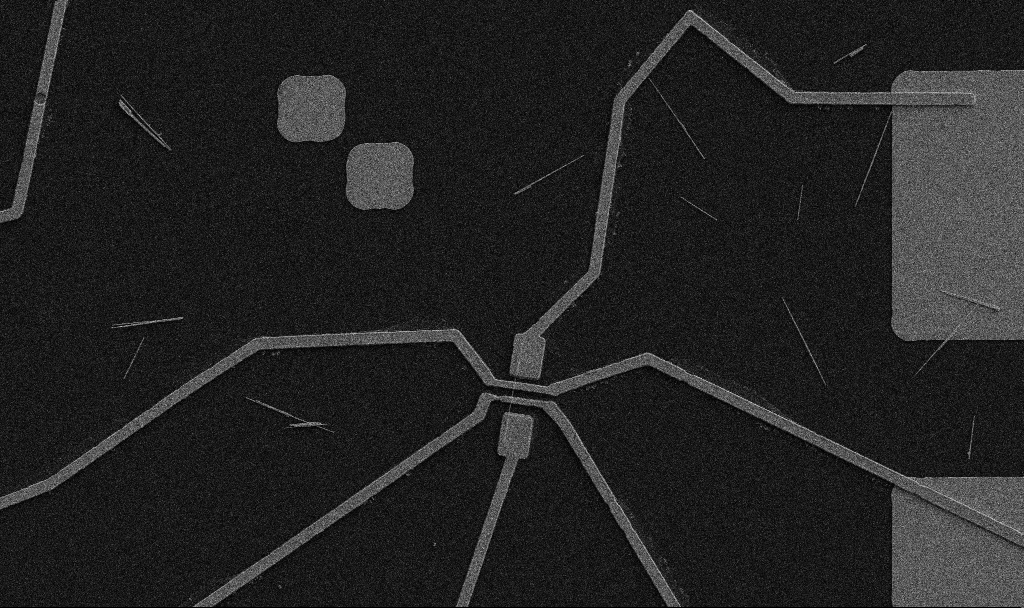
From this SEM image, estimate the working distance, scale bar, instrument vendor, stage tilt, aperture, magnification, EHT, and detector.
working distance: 10.7 mm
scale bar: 10000 nm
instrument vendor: Zeiss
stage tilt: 0°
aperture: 30 µm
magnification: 5 K X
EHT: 5 kV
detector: SE2